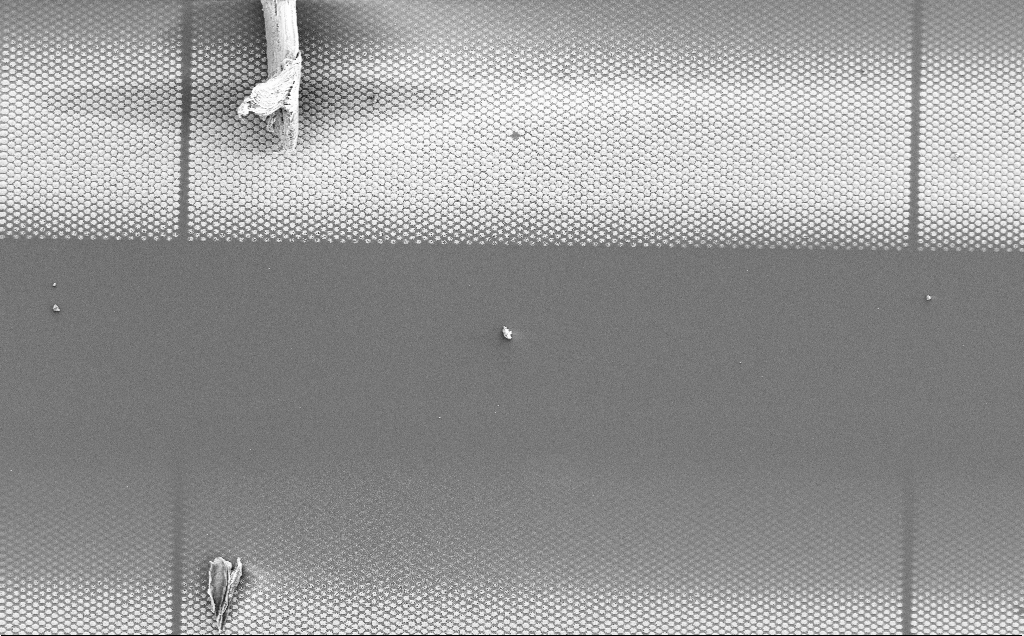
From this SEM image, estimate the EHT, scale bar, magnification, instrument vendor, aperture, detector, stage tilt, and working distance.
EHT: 5 kV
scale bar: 20000 nm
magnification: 0.885 K X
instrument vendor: Zeiss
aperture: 30 µm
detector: SE2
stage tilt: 0°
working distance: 20 mm